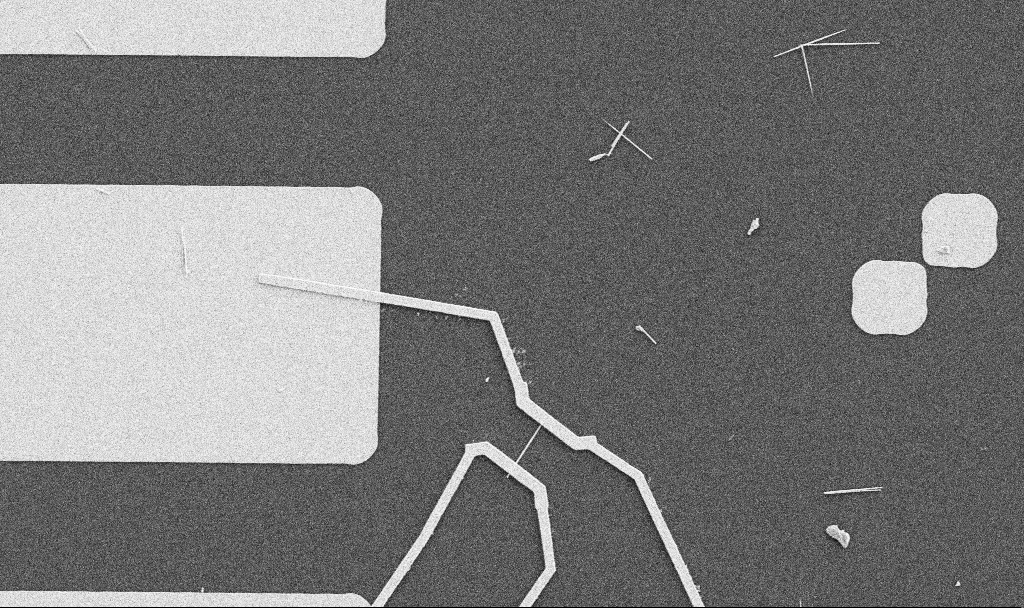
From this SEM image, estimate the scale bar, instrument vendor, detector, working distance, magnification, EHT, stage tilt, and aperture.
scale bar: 10000 nm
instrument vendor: Zeiss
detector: SE2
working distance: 10.7 mm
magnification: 5 K X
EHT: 5 kV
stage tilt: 0°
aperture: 30 µm